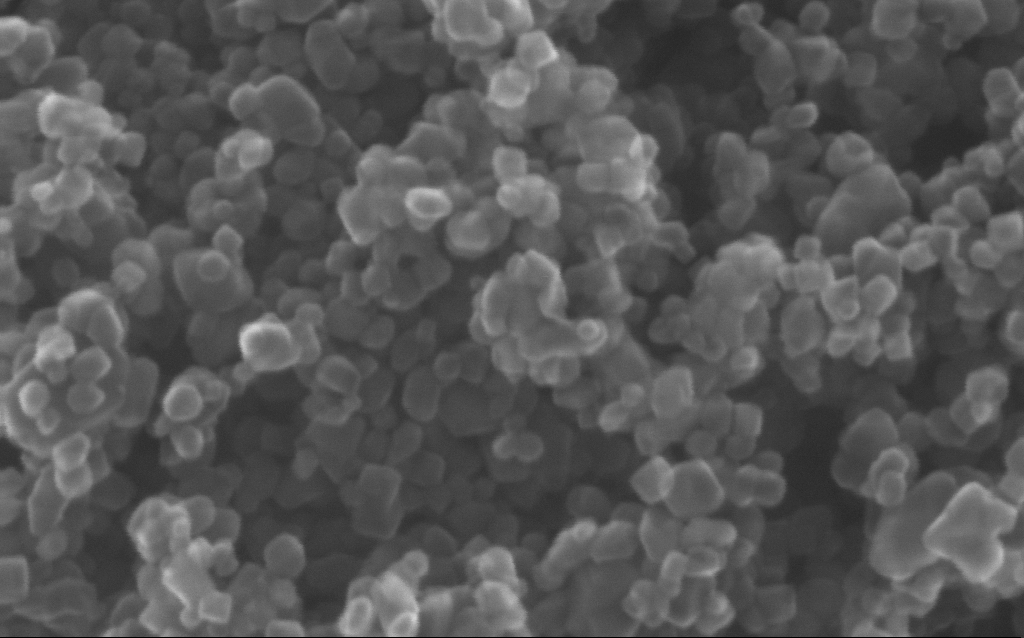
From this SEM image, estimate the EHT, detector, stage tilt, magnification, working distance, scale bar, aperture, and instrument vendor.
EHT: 20 kV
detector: InLens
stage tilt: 0°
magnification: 600 K X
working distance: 3 mm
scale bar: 100 nm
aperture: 30 µm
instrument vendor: Zeiss